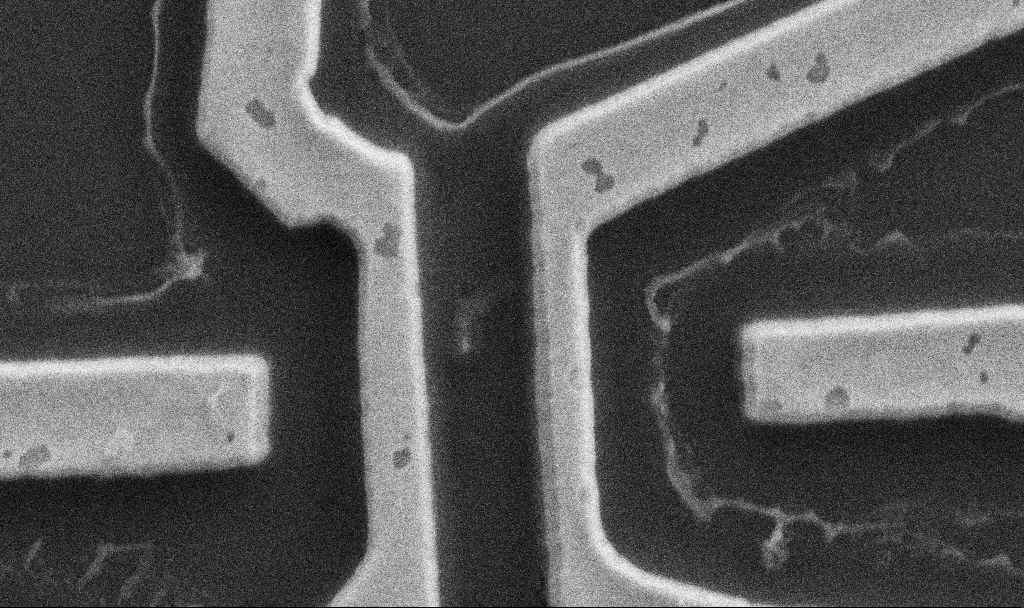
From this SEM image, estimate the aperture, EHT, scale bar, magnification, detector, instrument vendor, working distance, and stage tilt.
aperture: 30 µm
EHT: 5 kV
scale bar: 1000 nm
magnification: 60 K X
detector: SE2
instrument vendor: Zeiss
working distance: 10.7 mm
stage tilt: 0°